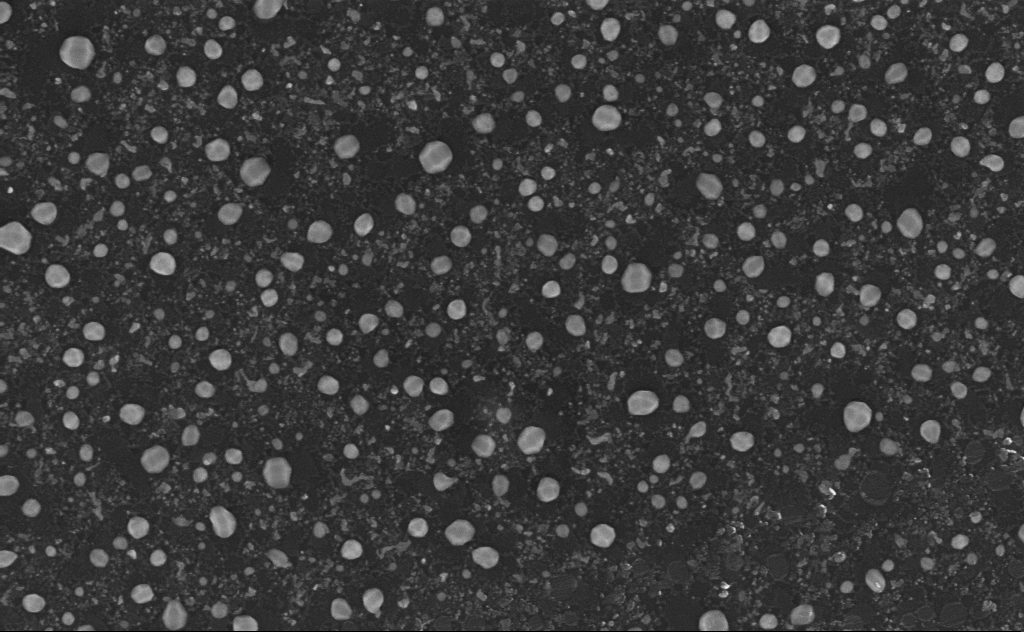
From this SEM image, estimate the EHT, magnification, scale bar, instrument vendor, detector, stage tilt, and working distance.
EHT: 5 kV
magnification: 80 K X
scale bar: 200 nm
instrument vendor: Zeiss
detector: InLens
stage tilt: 0°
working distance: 4 mm